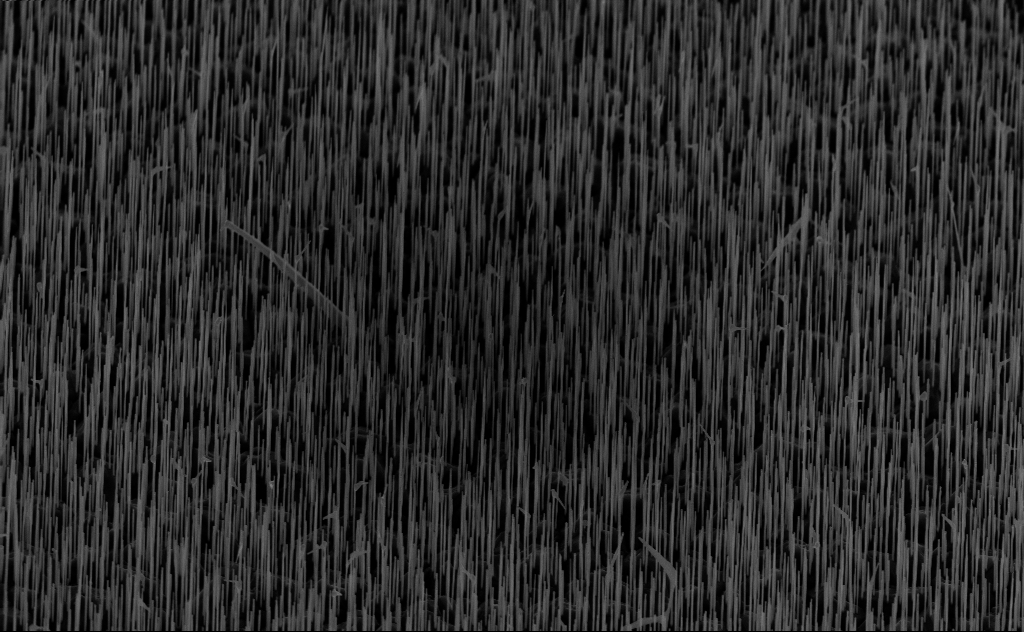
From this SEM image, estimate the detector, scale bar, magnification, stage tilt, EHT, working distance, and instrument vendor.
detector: InLens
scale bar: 2000 nm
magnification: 10 K X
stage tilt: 45°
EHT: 10 kV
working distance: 6 mm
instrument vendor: Zeiss